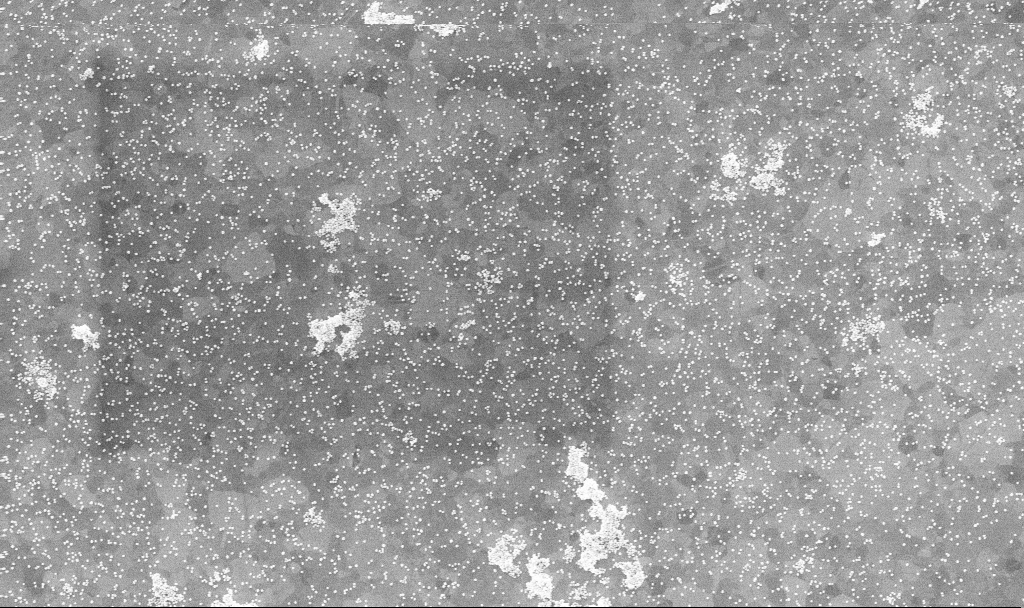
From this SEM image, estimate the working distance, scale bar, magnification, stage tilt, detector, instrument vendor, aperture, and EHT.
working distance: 3.3 mm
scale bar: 1000 nm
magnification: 50 K X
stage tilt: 0°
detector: InLens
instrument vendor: Zeiss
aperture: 30 µm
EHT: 10 kV